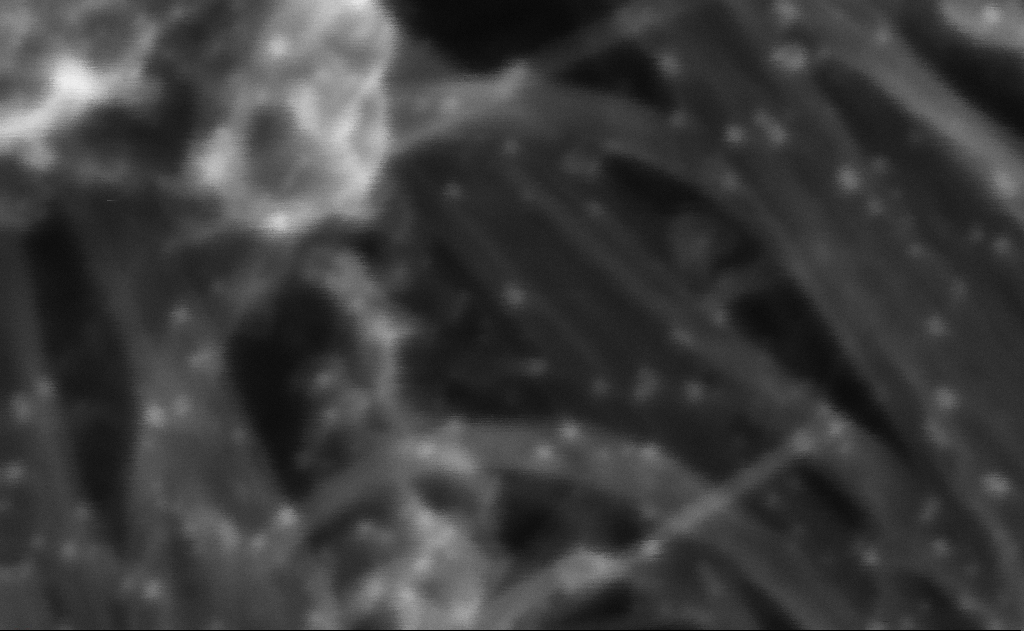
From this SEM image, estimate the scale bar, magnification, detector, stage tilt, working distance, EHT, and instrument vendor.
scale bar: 20 nm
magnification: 2068.94 K X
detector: InLens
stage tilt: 0°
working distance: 3 mm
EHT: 10 kV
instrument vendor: Zeiss